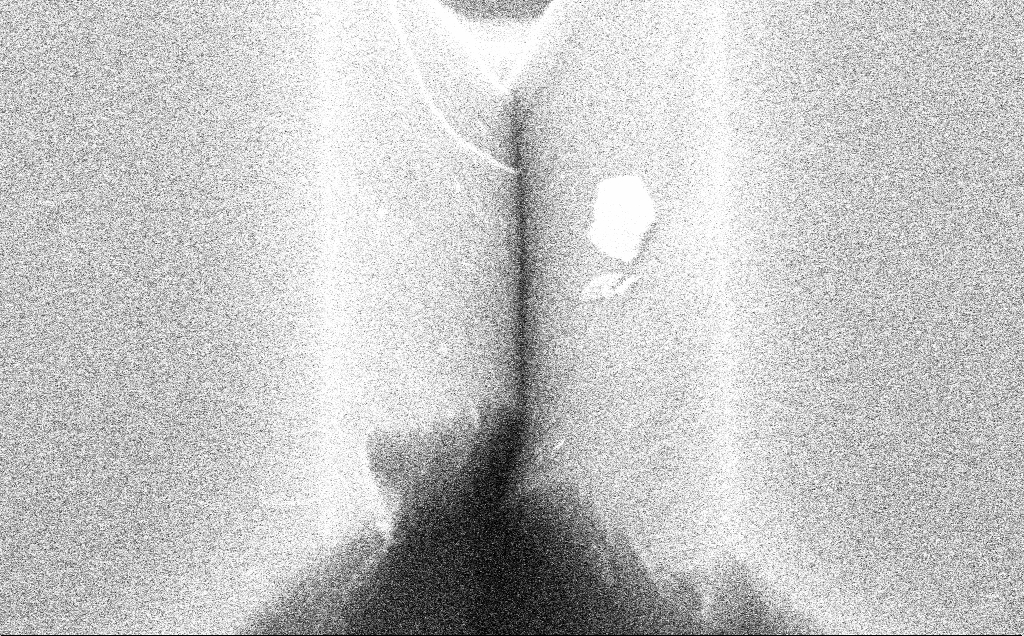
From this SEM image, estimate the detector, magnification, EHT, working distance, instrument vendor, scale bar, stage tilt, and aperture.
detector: SE2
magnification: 66.07 K X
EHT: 10 kV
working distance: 10 mm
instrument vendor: Zeiss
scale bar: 1000 nm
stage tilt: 50°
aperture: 30 µm